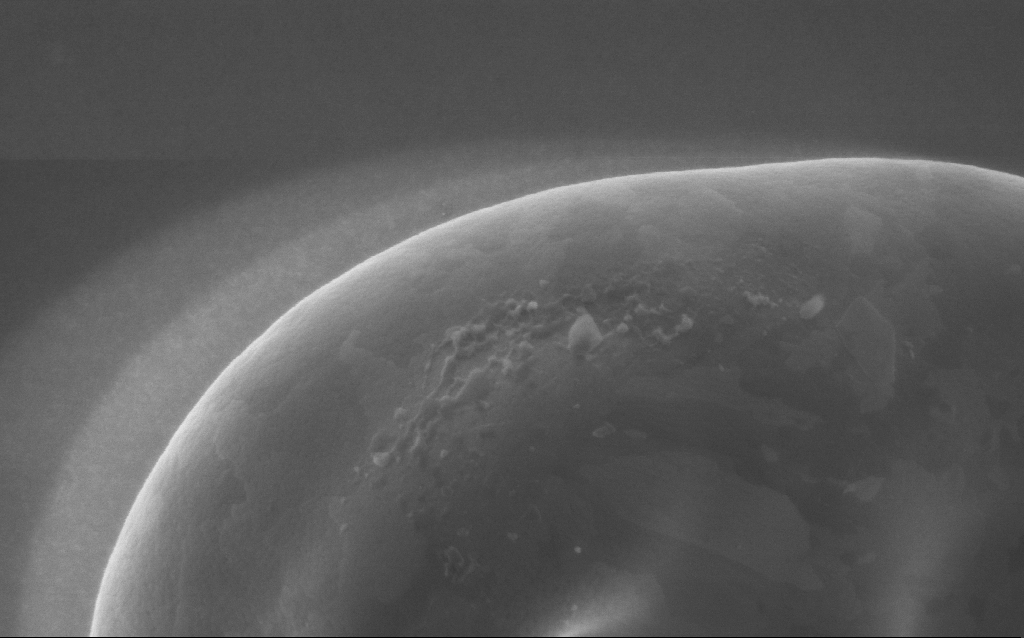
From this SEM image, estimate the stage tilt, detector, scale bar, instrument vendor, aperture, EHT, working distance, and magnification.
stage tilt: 0°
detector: InLens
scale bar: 200 nm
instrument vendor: Zeiss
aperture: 30 µm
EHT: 5 kV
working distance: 4 mm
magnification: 100 K X